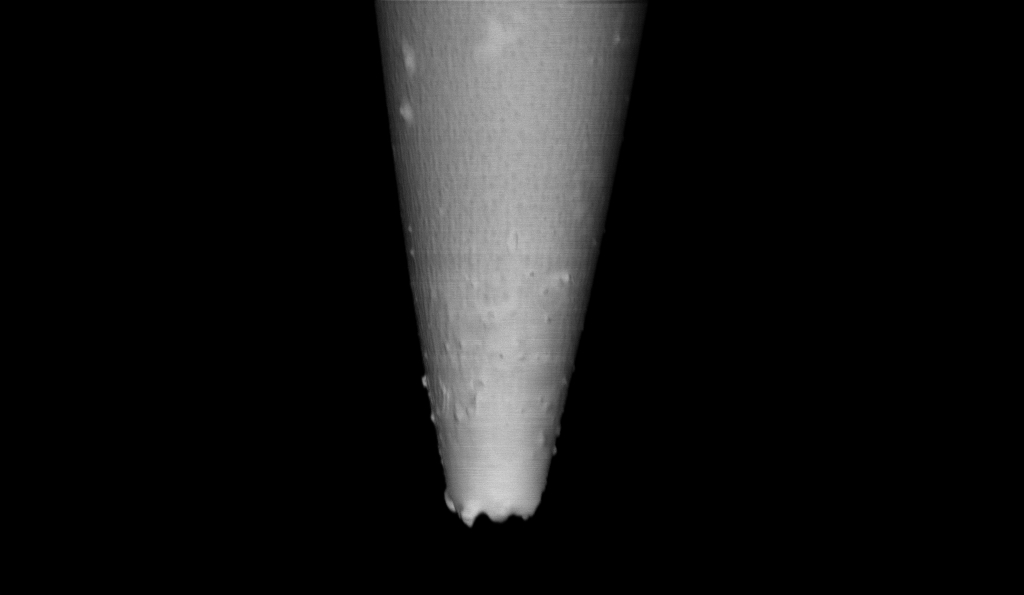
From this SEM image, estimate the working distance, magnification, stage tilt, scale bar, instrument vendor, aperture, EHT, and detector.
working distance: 6.8 mm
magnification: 15 K X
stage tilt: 0°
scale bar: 1000 nm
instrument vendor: Zeiss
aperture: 30 µm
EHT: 1 kV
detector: InLens